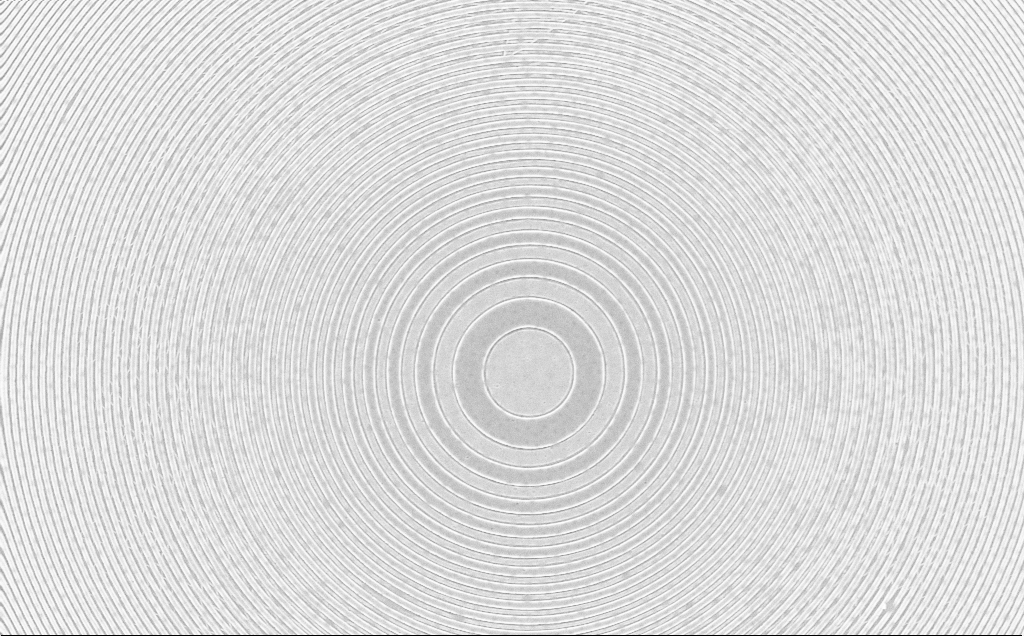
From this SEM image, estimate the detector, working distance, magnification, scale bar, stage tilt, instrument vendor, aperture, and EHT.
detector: InLens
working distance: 6 mm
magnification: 4.57 K X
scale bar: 10000 nm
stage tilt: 0°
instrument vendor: Zeiss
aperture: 30 µm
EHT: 5 kV